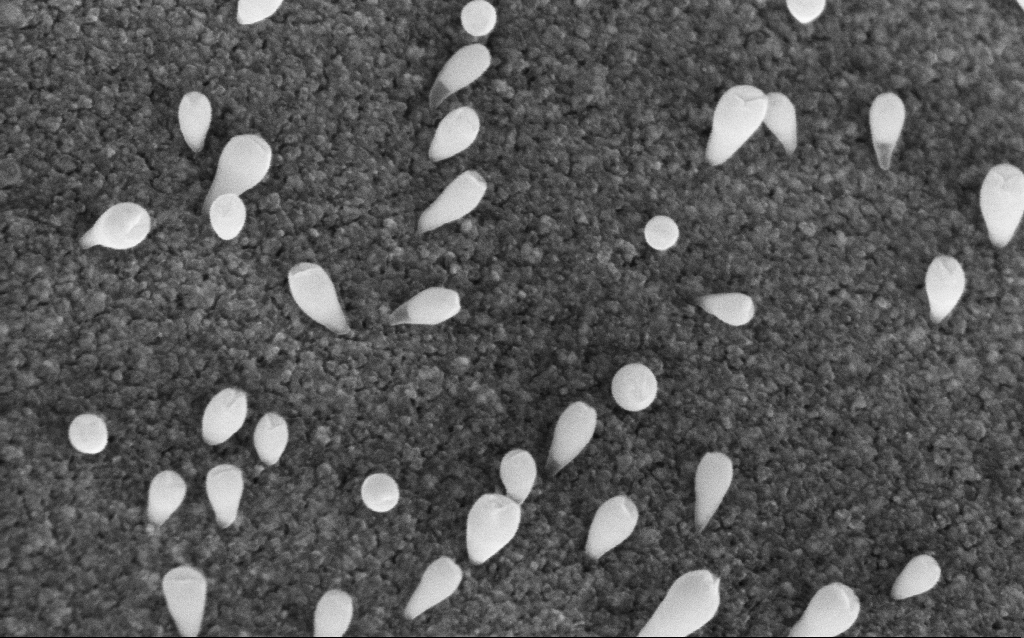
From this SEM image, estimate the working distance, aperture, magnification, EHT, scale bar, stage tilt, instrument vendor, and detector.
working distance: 6 mm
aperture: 30 µm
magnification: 163.95 K X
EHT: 5 kV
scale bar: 200 nm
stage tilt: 42.7°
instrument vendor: Zeiss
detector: InLens